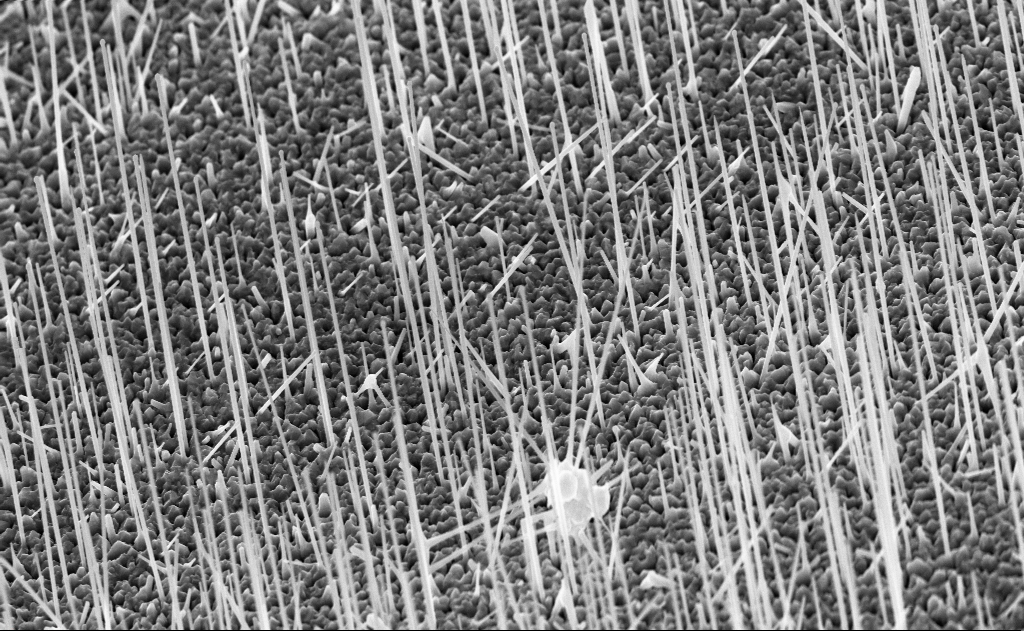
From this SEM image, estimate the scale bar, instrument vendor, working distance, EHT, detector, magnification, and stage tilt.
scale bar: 2000 nm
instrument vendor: Zeiss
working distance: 8 mm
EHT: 10 kV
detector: InLens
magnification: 20 K X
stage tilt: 0°